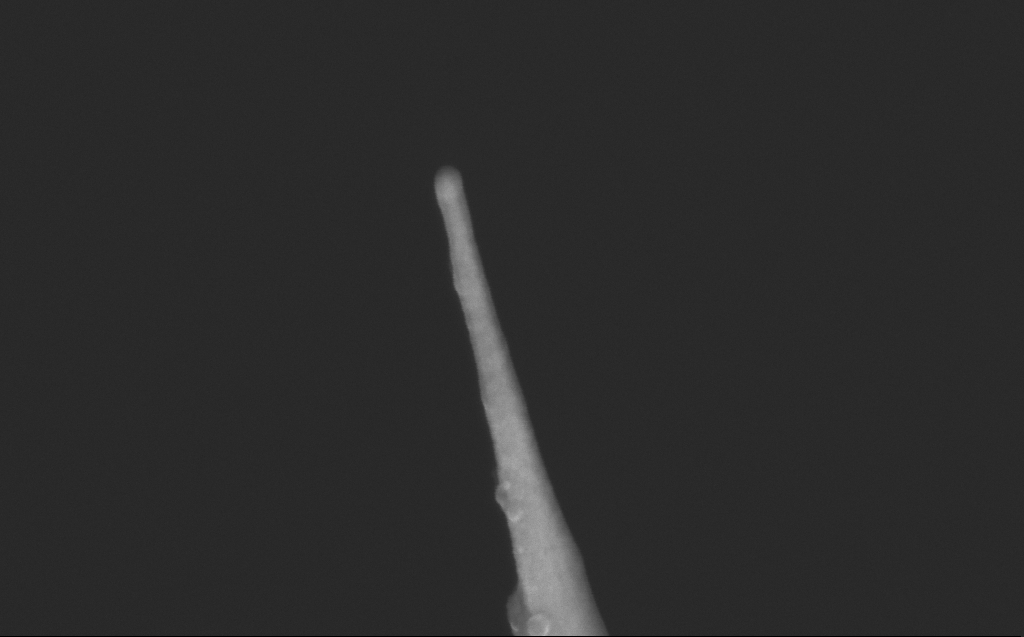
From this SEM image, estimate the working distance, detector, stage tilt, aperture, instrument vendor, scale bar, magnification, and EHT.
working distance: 6 mm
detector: InLens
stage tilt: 40°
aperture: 30 µm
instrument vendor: Zeiss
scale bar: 100 nm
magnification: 178.61 K X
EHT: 10 kV